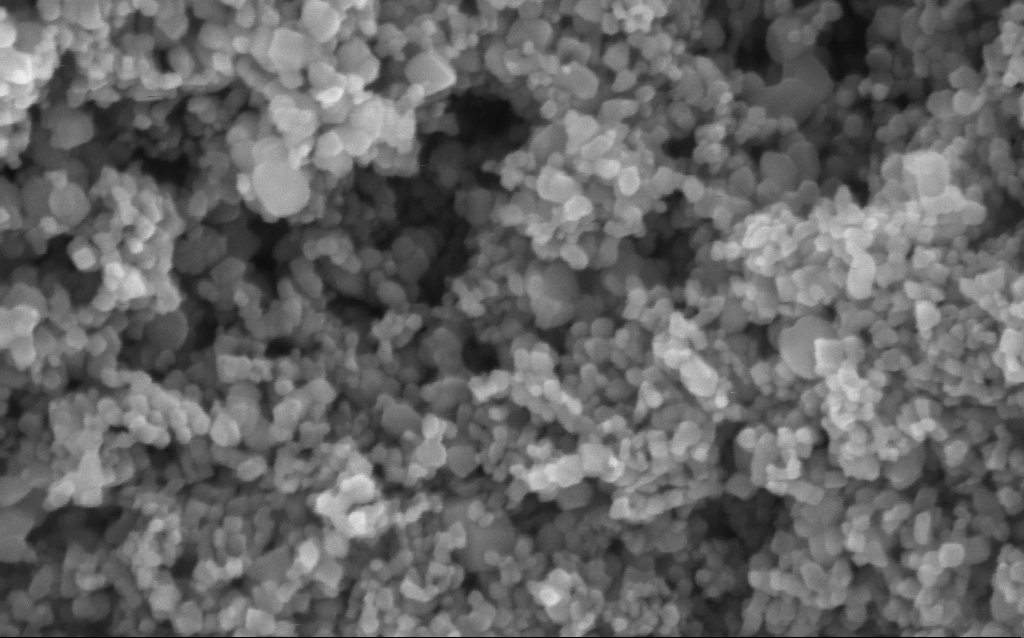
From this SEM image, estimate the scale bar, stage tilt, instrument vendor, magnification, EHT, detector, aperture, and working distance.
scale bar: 200 nm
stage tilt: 0°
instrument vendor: Zeiss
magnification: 290.6 K X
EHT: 5 kV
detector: InLens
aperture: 30 µm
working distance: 4.7 mm